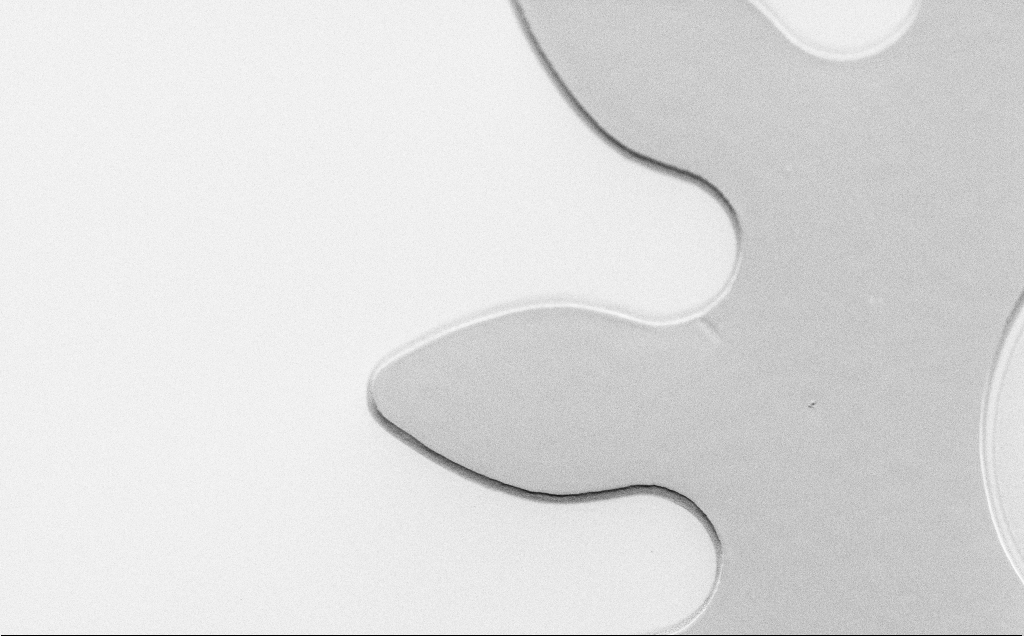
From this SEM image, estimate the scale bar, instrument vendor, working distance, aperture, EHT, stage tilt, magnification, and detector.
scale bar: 20000 nm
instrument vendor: Zeiss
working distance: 7 mm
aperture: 30 µm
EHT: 1.5 kV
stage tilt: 45°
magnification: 1.46 K X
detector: SE2